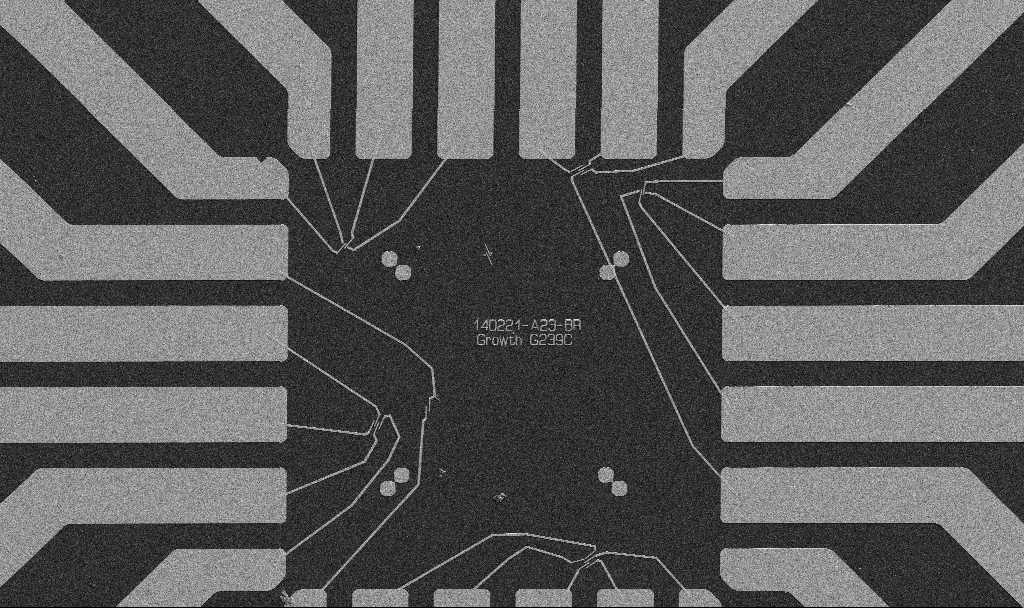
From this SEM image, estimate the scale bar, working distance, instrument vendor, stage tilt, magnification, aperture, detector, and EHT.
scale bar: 20000 nm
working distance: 10.7 mm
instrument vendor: Zeiss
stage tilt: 0°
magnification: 1 K X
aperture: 30 µm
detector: SE2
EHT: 5 kV